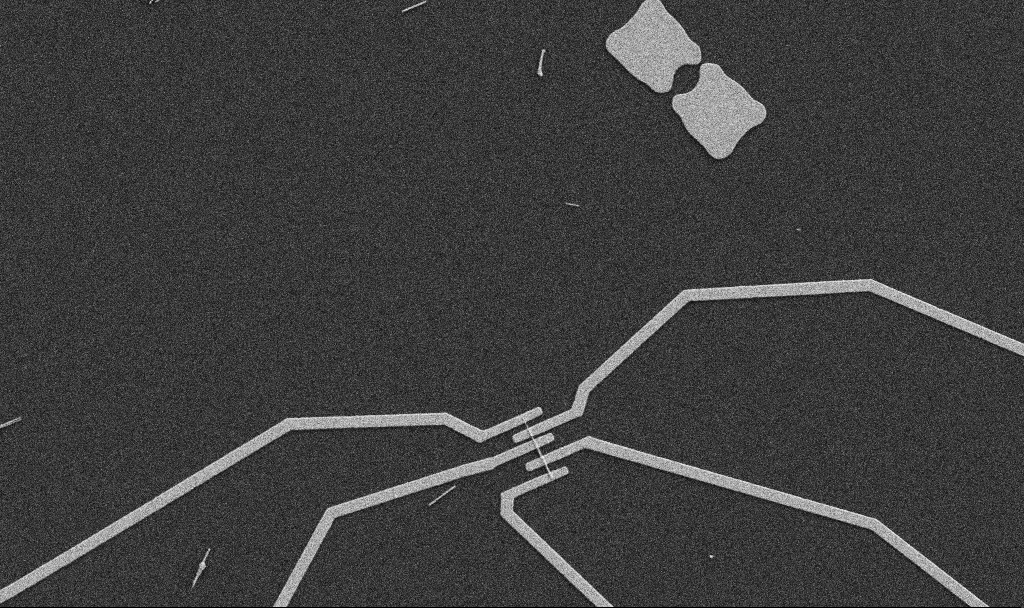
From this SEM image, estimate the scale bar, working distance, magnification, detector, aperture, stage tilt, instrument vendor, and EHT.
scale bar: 10000 nm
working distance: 10.7 mm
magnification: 5 K X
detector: SE2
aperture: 30 µm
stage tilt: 0°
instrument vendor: Zeiss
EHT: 5 kV